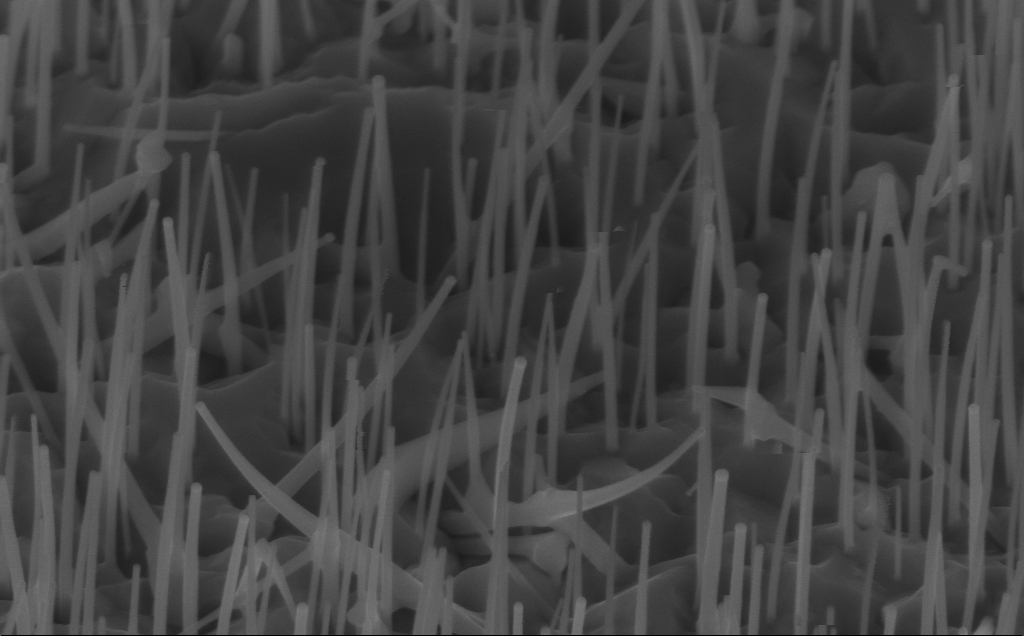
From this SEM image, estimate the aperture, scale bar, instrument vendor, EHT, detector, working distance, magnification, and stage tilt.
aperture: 30 µm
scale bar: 200 nm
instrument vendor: Zeiss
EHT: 10 kV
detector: InLens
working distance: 7 mm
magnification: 80 K X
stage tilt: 45°